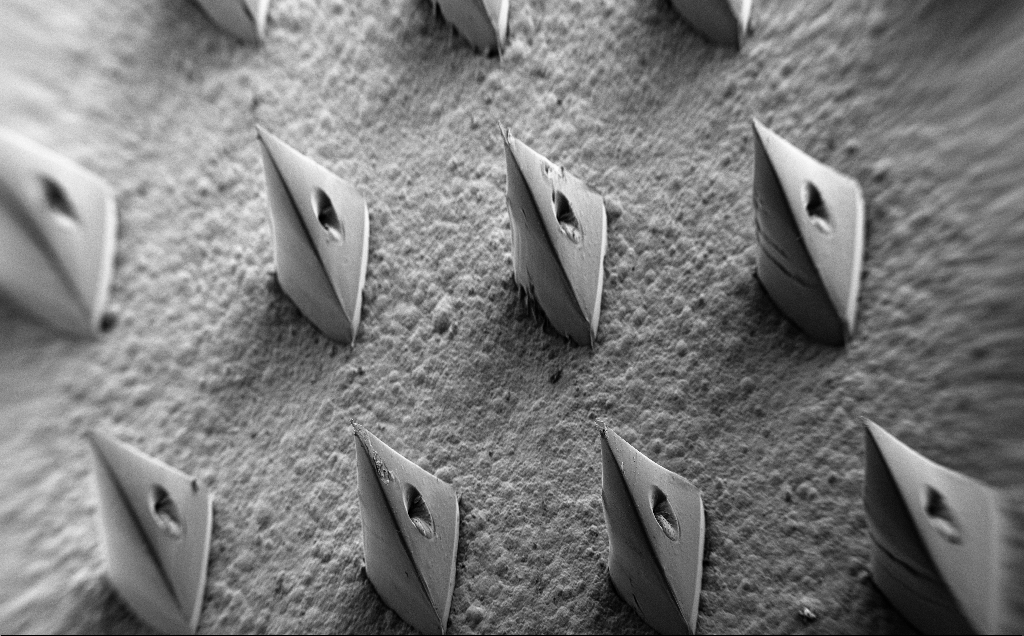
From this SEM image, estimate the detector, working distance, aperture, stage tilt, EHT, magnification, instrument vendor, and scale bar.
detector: SE2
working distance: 11 mm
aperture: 30 µm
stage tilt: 35°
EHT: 5 kV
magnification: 0.061 K X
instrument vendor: Zeiss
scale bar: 1e+06 nm